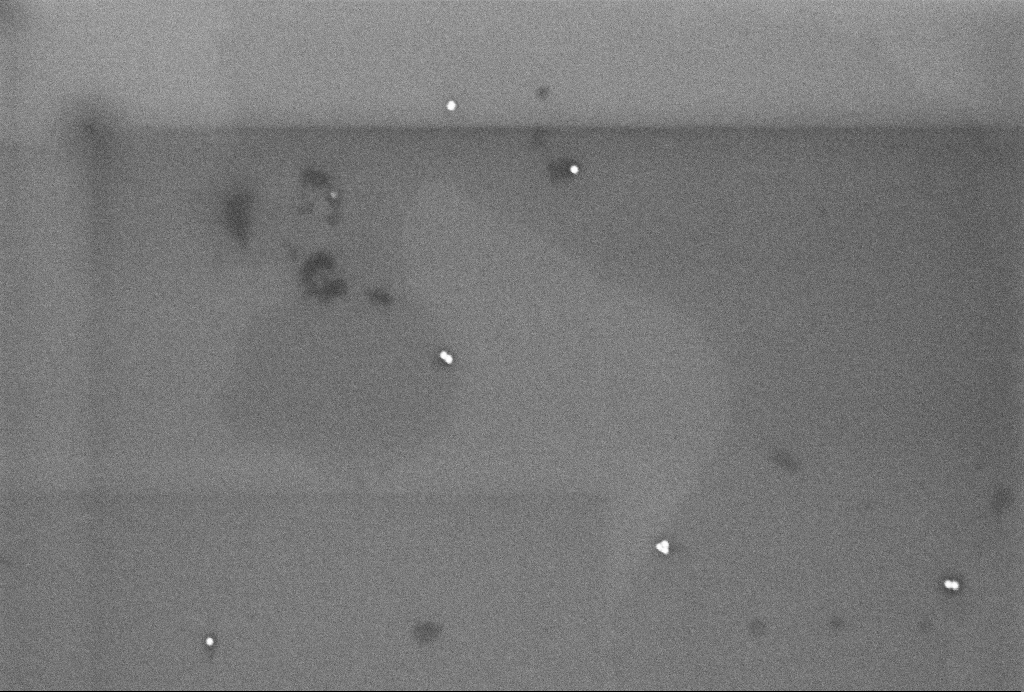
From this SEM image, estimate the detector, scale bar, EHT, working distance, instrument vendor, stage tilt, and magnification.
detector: InLens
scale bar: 200 nm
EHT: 3 kV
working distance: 3.2 mm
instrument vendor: Zeiss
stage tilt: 0°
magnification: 102.77 K X